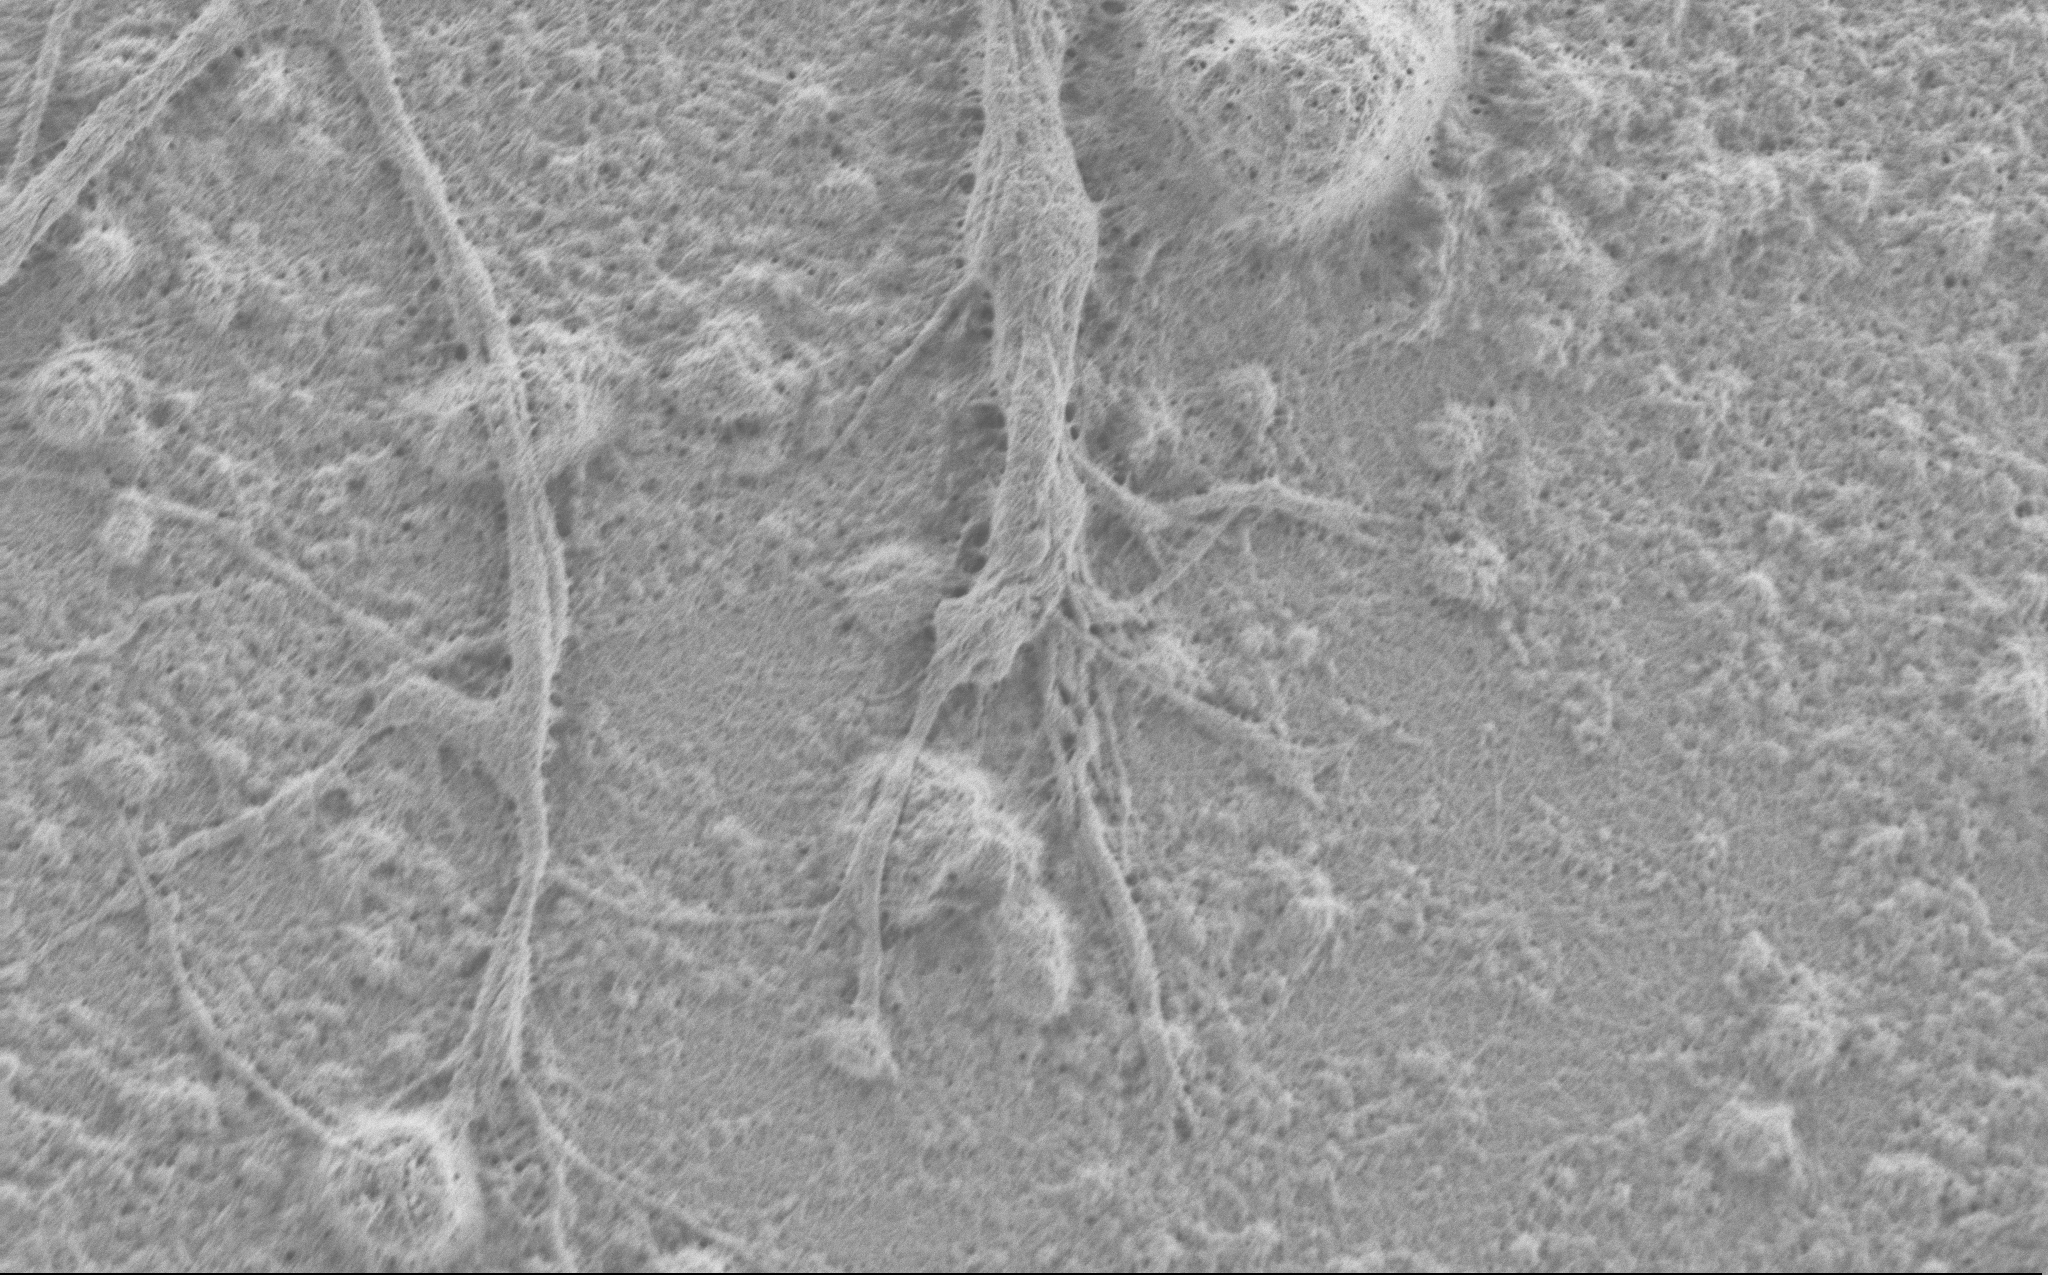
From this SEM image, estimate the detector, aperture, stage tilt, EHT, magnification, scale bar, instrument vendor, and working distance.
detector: SE2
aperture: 30 µm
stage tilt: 0°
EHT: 0.9 kV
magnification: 10 K X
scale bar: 2000 nm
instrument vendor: Zeiss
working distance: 4 mm